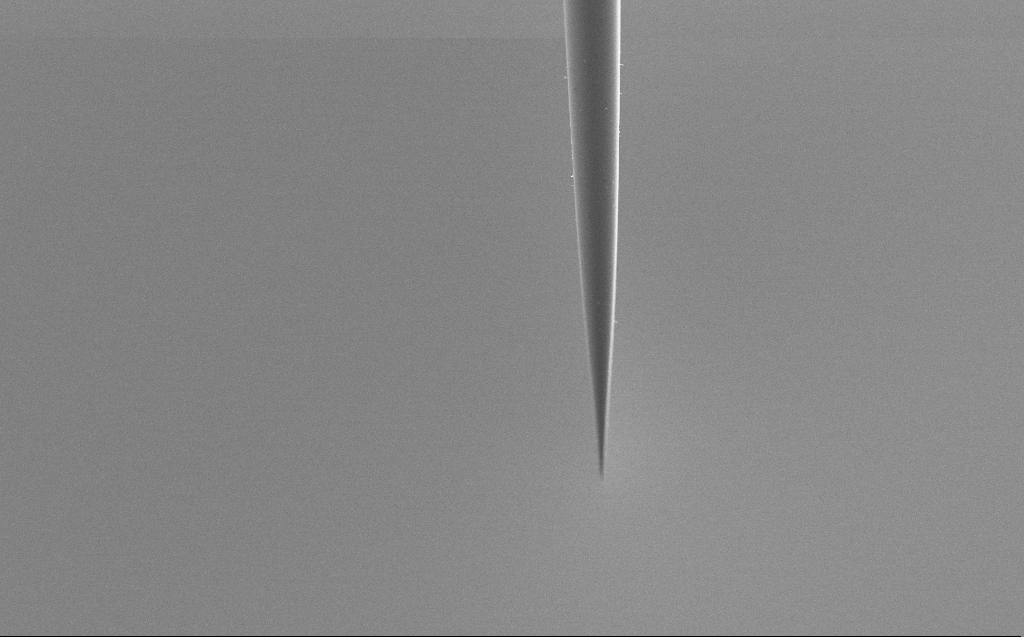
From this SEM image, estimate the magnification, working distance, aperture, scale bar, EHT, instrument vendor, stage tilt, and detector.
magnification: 0.956 K X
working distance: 4 mm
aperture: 30 µm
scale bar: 20000 nm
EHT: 2 kV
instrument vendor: Zeiss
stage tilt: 45°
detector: SE2